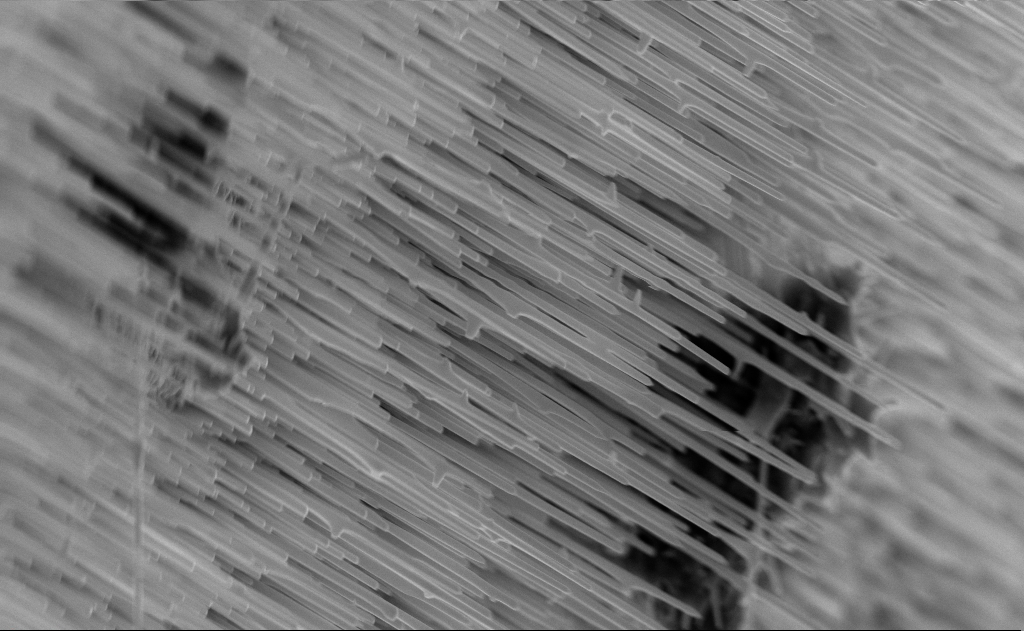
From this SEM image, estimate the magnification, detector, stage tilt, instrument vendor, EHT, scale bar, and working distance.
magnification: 20 K X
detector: InLens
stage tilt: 0°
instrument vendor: Zeiss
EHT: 10 kV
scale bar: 2000 nm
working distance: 6 mm